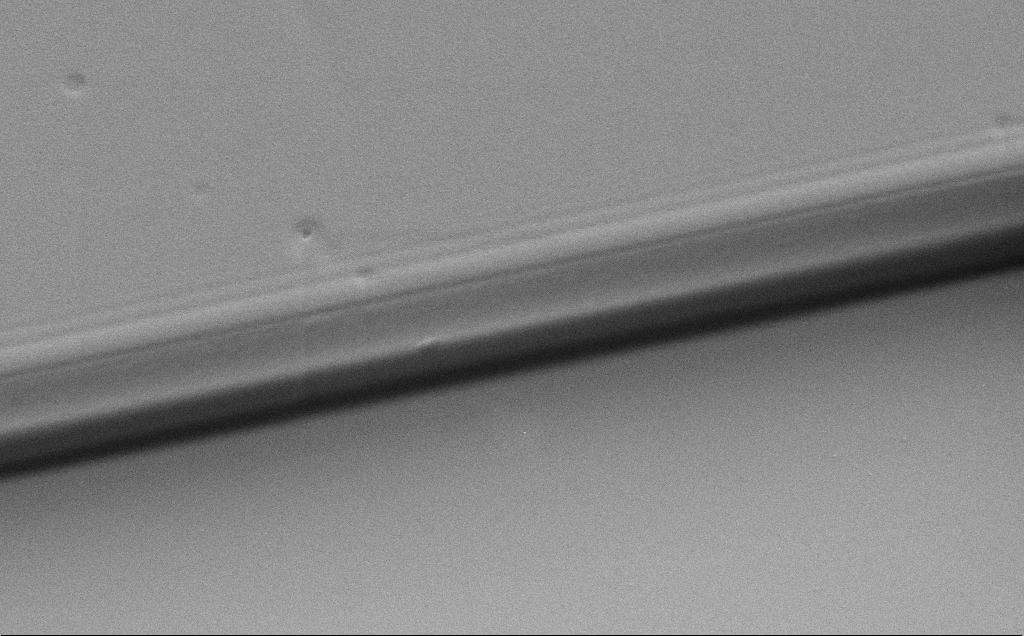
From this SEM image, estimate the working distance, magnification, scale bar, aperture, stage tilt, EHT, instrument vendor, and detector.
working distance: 11 mm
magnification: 6.77 K X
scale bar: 10000 nm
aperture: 30 µm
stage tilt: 30°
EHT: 5 kV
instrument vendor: Zeiss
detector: SE2